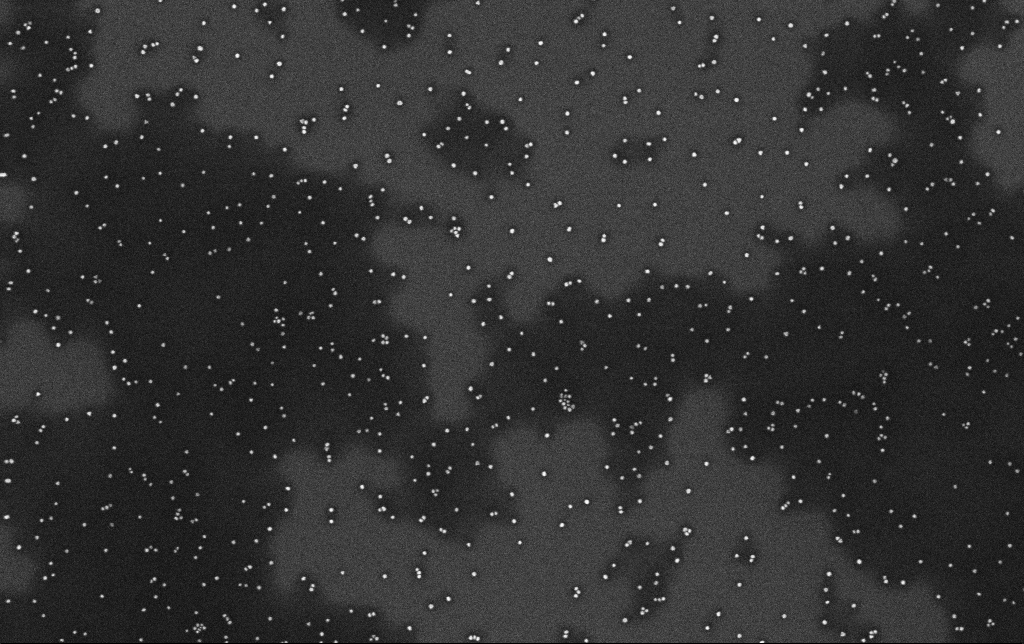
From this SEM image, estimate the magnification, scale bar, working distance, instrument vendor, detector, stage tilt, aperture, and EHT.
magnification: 100 K X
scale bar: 200 nm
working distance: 3.3 mm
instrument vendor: Zeiss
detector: InLens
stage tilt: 0°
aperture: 30 µm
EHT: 10 kV